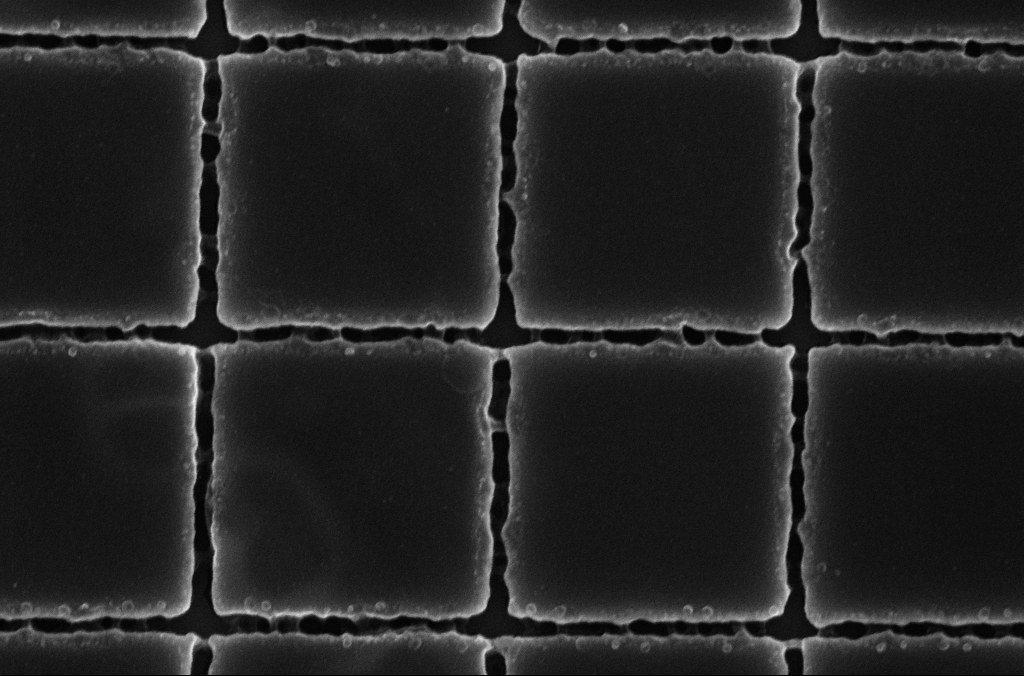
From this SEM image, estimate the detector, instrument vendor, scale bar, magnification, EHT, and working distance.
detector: InLens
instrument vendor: Zeiss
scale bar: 1000 nm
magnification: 55.18 K X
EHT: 5 kV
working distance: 3.9 mm